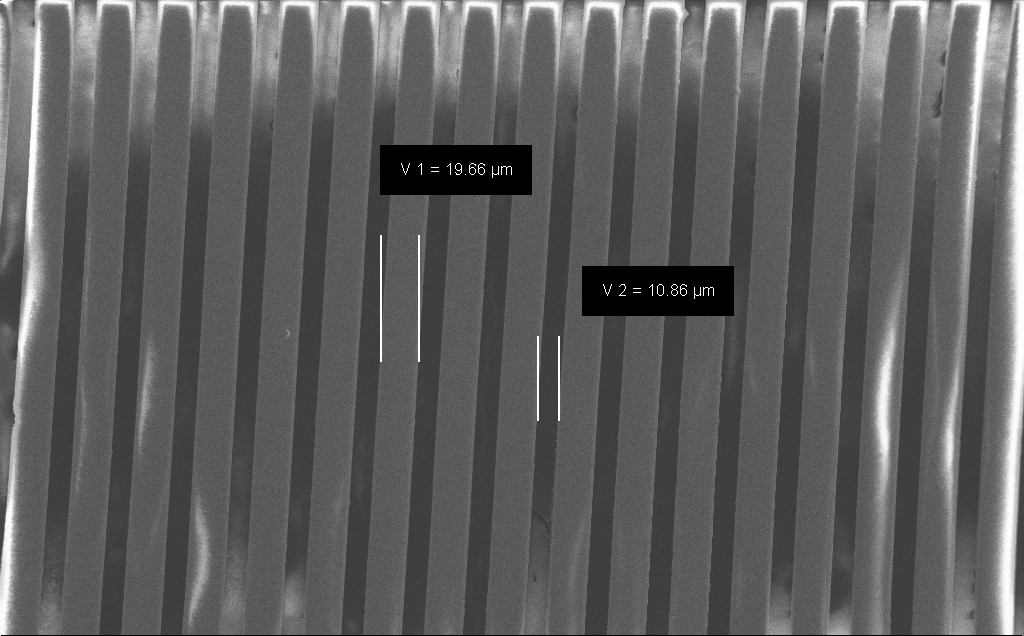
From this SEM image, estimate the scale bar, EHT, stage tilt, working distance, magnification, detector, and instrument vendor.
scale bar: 100000 nm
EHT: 1.2 kV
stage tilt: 0°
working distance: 5 mm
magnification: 0.71 K X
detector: SE2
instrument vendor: Zeiss